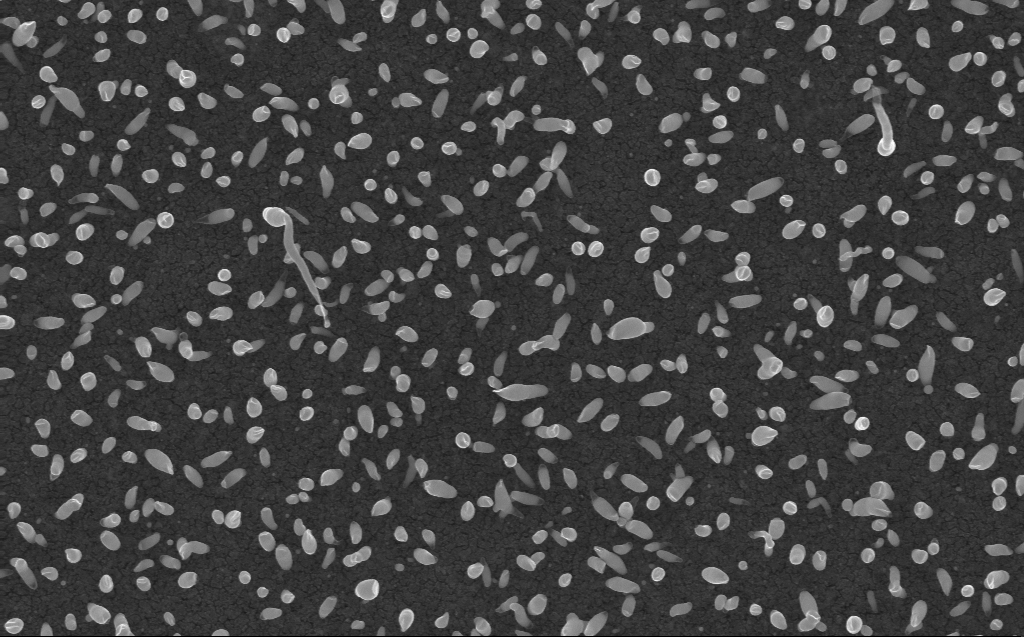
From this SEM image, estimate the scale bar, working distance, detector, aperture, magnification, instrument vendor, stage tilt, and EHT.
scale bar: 1000 nm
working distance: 3 mm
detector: InLens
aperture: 30 µm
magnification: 50 K X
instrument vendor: Zeiss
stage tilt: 0°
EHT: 10 kV